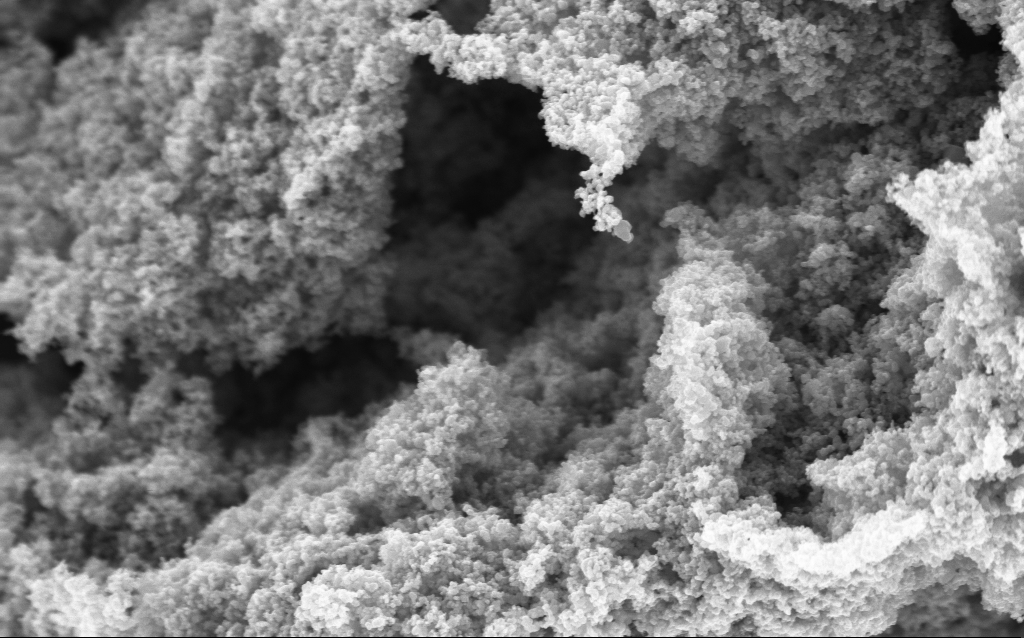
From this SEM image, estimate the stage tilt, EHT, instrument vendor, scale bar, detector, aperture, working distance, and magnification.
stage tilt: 0°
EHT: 5 kV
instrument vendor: Zeiss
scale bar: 1000 nm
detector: InLens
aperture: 30 µm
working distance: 4.4 mm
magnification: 68.66 K X